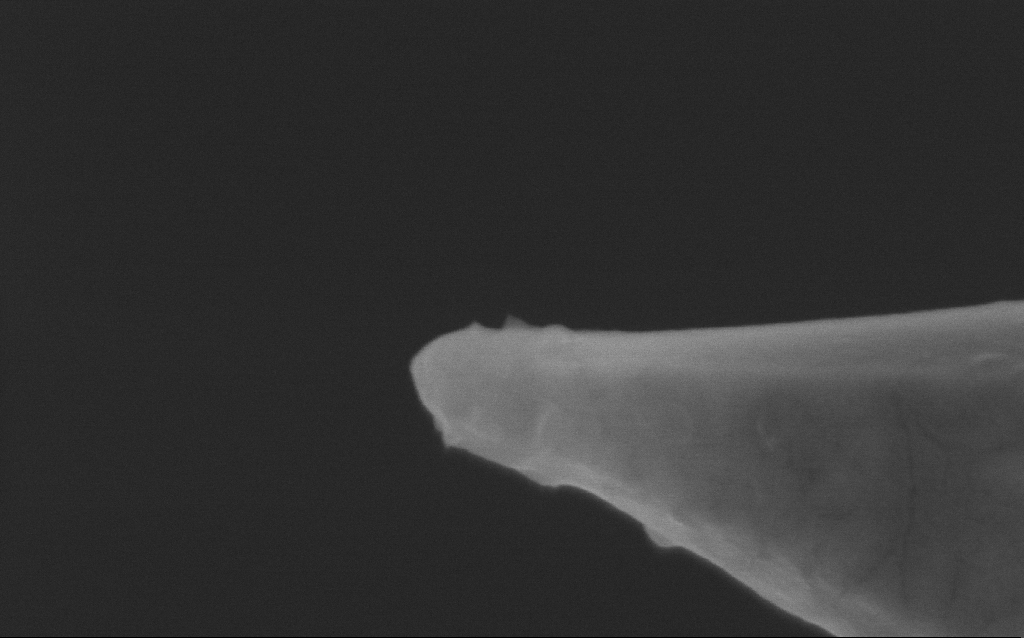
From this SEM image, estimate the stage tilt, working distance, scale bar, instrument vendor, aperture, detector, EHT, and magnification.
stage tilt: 0°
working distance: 5 mm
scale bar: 200 nm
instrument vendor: Zeiss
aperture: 30 µm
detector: InLens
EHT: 5 kV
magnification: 250 K X